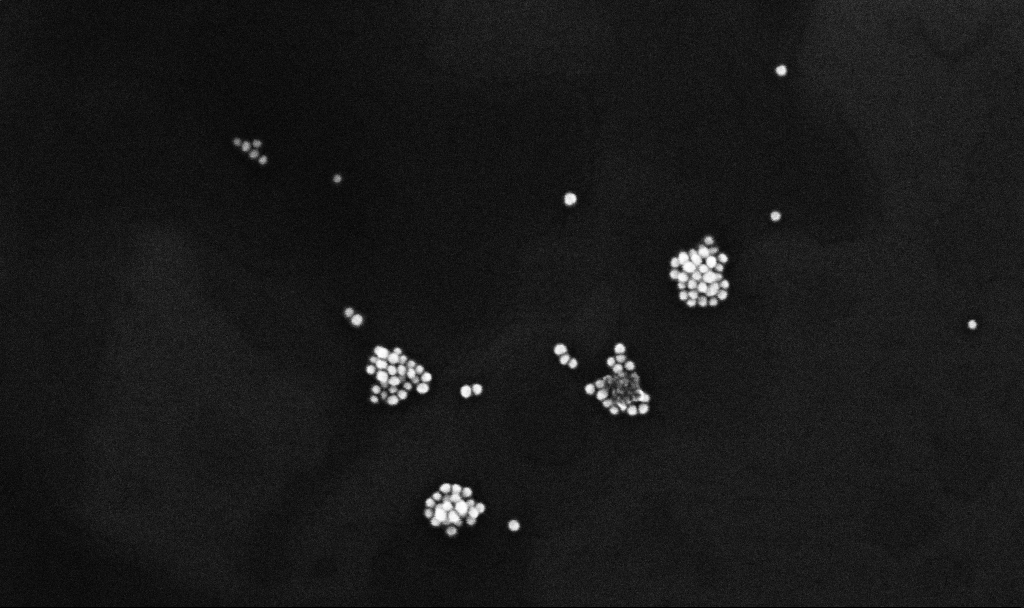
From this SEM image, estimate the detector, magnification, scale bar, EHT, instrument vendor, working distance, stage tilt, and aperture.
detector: InLens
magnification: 179.87 K X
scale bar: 200 nm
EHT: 10 kV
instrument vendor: Zeiss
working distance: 3.3 mm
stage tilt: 0°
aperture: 30 µm